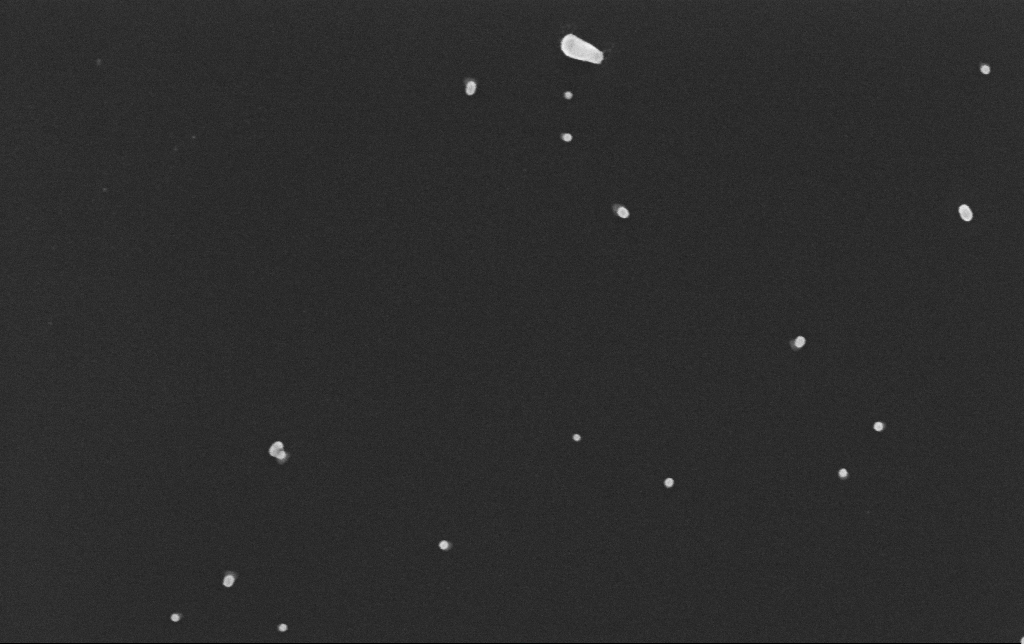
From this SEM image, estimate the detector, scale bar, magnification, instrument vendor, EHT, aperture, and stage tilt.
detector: InLens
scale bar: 200 nm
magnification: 150 K X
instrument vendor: Zeiss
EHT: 10 kV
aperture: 30 µm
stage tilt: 0°